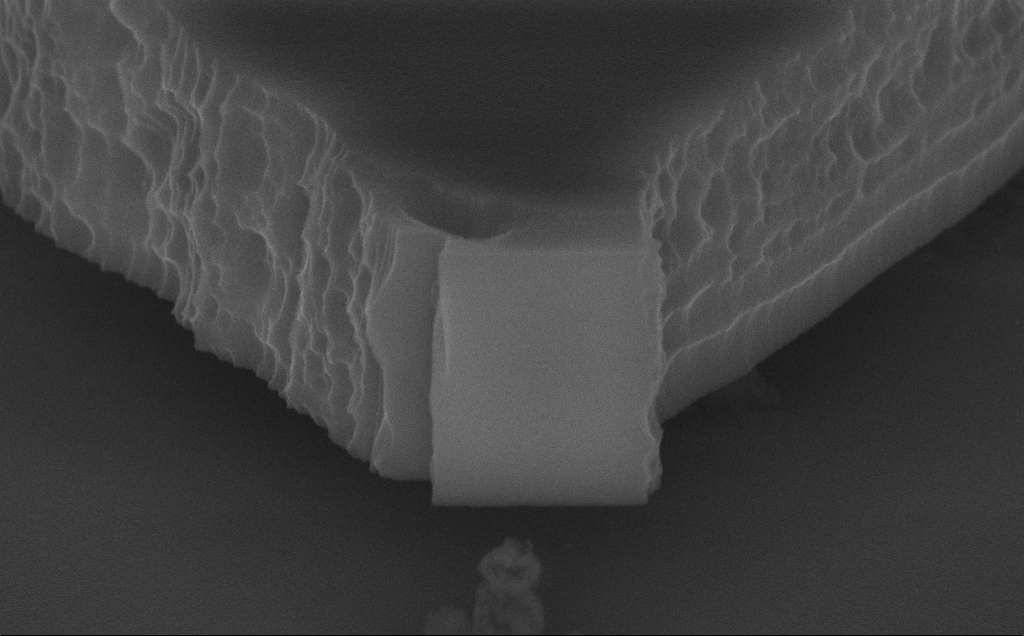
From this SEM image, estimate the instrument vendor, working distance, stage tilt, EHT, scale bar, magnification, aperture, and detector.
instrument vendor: Zeiss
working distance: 9 mm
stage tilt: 70°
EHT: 10 kV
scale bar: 1000 nm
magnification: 44.97 K X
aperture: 30 µm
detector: InLens